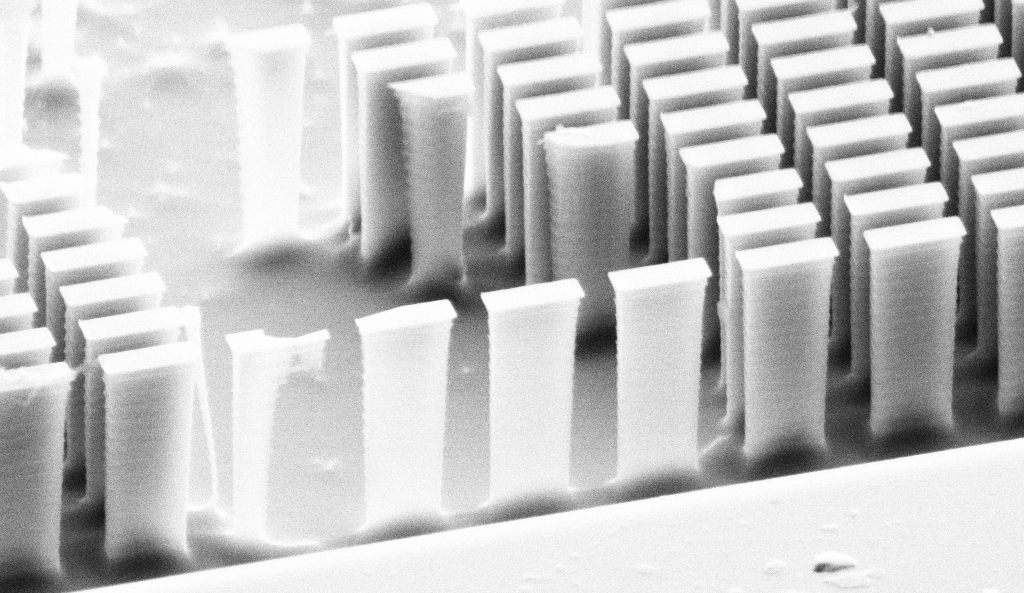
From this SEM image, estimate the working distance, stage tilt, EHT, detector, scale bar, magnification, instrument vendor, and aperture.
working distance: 8 mm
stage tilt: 62°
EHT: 10 kV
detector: SE2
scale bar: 2000 nm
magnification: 12.27 K X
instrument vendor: Zeiss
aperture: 30 µm